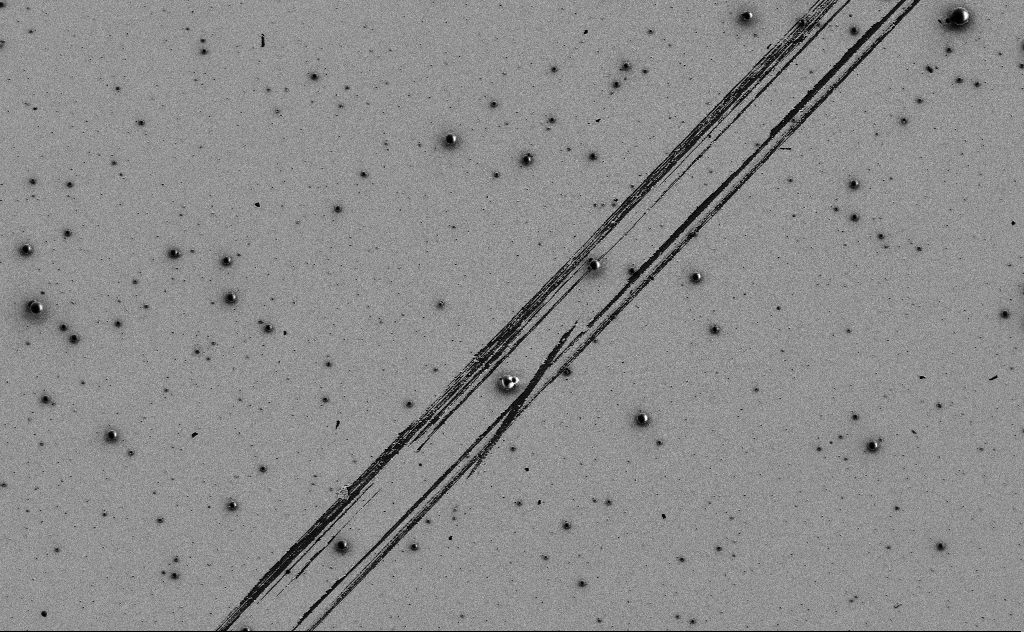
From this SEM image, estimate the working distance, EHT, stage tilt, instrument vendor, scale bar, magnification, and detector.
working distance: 10 mm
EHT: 3 kV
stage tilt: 0°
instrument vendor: Zeiss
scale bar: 10000 nm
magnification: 2.33 K X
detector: SE2